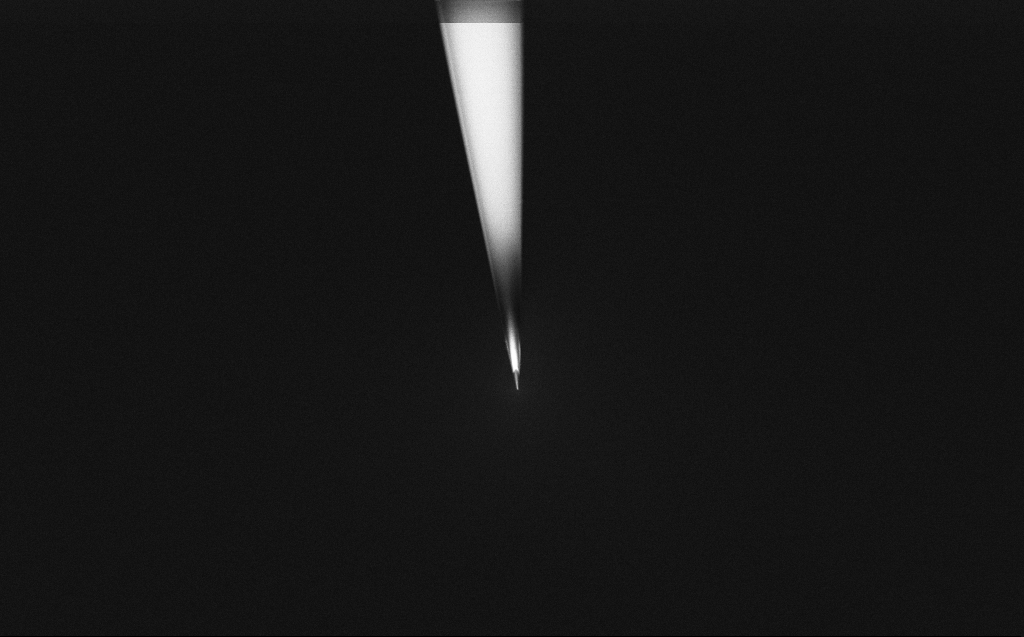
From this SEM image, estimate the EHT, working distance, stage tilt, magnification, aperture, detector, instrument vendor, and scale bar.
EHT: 2 kV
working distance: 6 mm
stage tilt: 45°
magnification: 2.5 K X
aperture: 30 µm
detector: InLens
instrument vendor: Zeiss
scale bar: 20000 nm